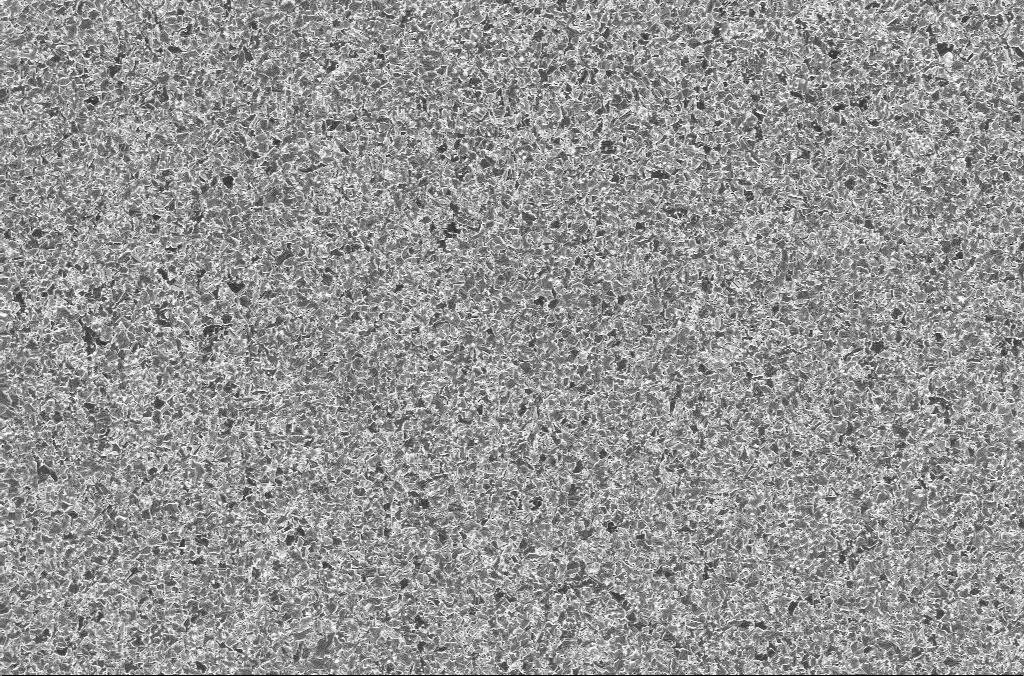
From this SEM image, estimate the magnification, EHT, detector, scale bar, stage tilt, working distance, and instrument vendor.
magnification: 5 K X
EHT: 2 kV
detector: InLens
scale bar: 10000 nm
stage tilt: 0°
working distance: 3 mm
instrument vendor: Zeiss